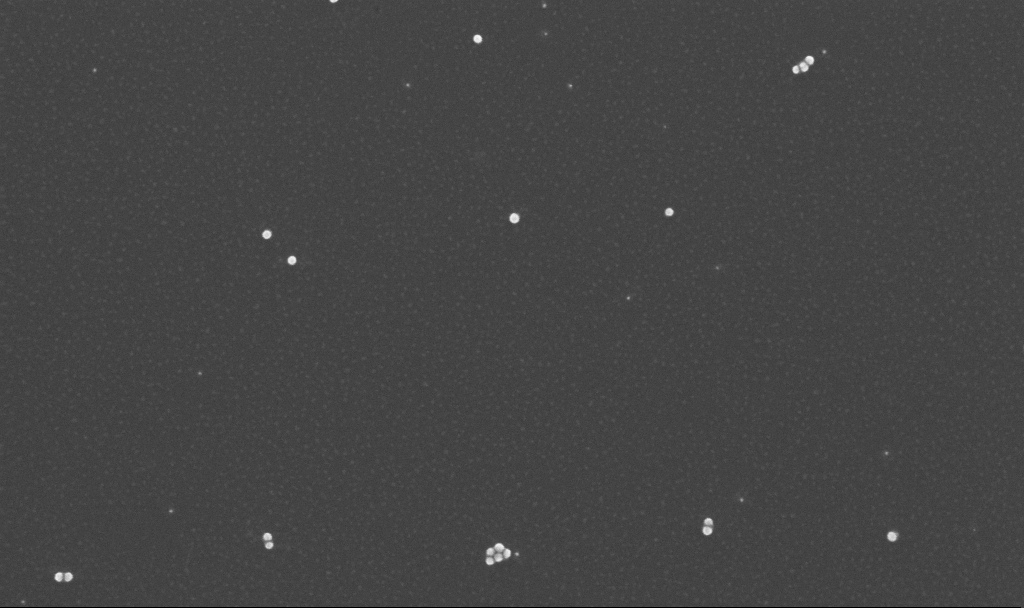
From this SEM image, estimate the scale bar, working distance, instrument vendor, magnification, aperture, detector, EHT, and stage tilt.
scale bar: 100 nm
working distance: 3.2 mm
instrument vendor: Zeiss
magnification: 150 K X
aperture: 30 µm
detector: InLens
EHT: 10 kV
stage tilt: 0°